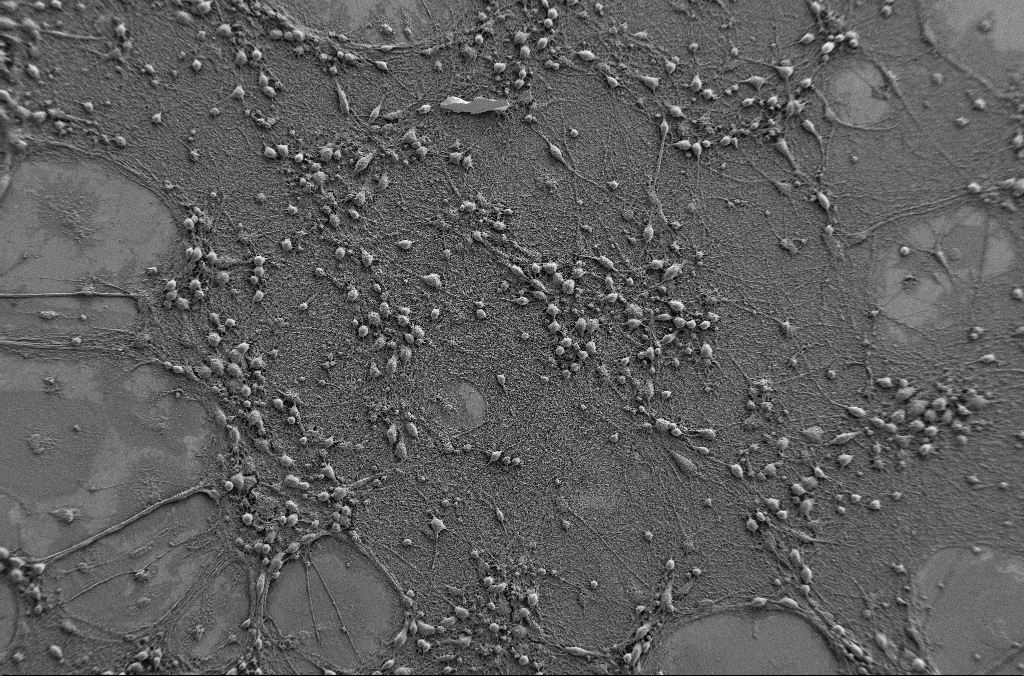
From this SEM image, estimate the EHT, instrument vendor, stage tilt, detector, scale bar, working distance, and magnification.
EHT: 1 kV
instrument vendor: Zeiss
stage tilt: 0°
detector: SE2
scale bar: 100000 nm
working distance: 4.1 mm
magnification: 0.5 K X